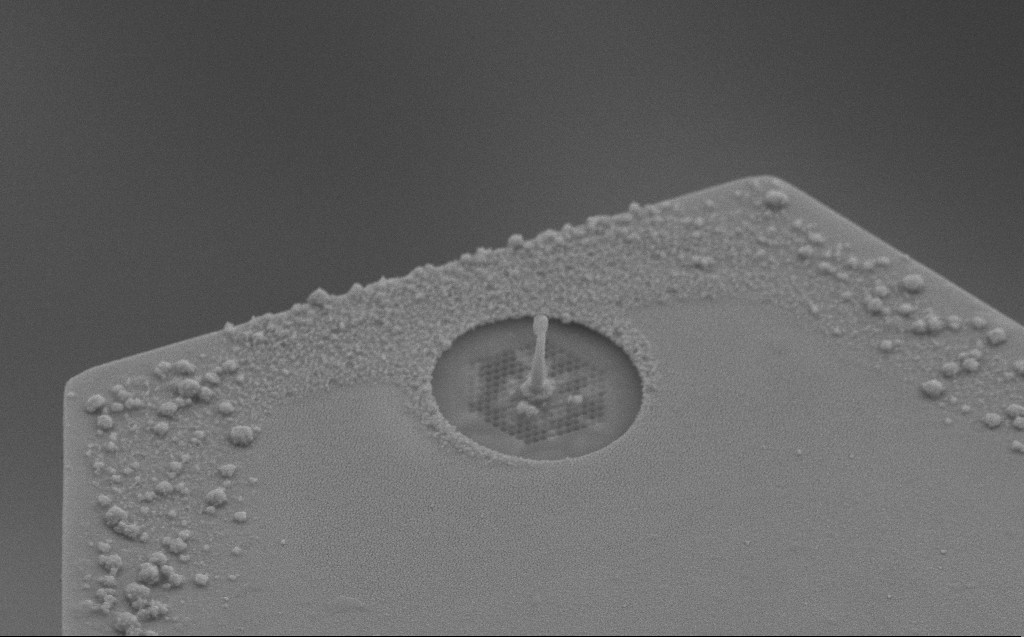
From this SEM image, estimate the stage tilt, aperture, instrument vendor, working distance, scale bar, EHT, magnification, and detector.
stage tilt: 45°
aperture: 30 µm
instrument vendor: Zeiss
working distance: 6 mm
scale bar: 2000 nm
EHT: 10 kV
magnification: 13.22 K X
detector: SE2